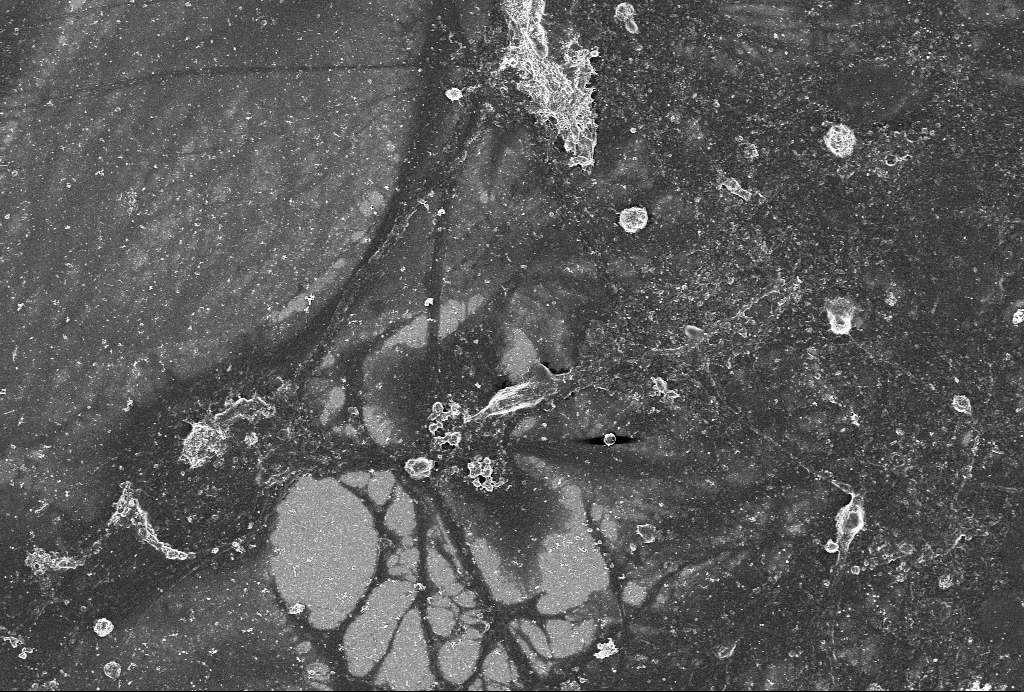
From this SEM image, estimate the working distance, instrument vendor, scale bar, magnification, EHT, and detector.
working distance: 6 mm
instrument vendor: Zeiss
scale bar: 10000 nm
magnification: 1.5 K X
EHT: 4 kV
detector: SE2